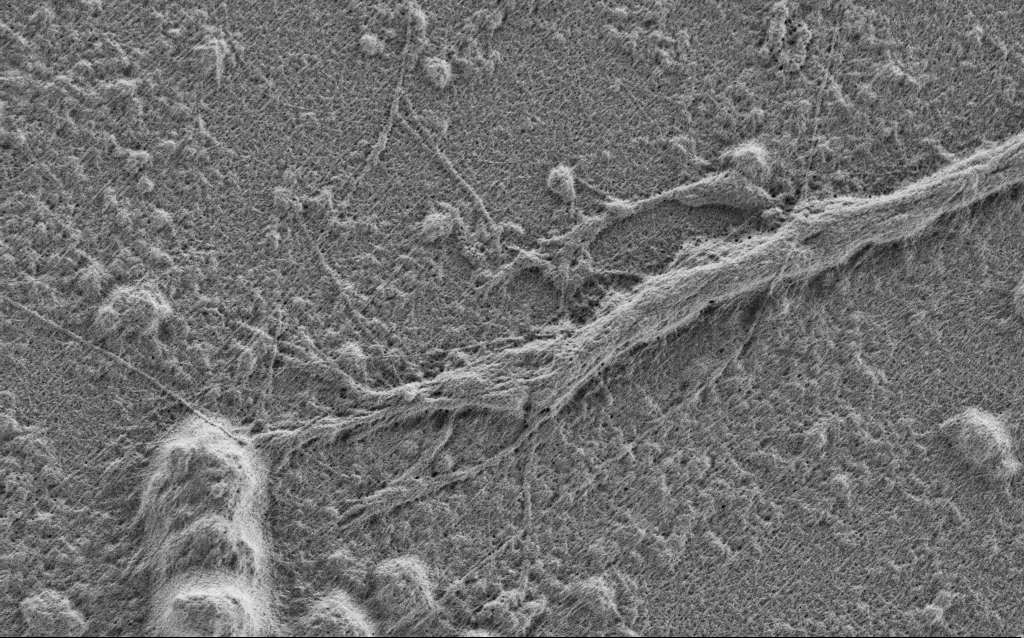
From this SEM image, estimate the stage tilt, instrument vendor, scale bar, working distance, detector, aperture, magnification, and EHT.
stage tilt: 0°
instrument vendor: Zeiss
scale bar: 2000 nm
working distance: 4 mm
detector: SE2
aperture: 30 µm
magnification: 10 K X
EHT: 0.9 kV